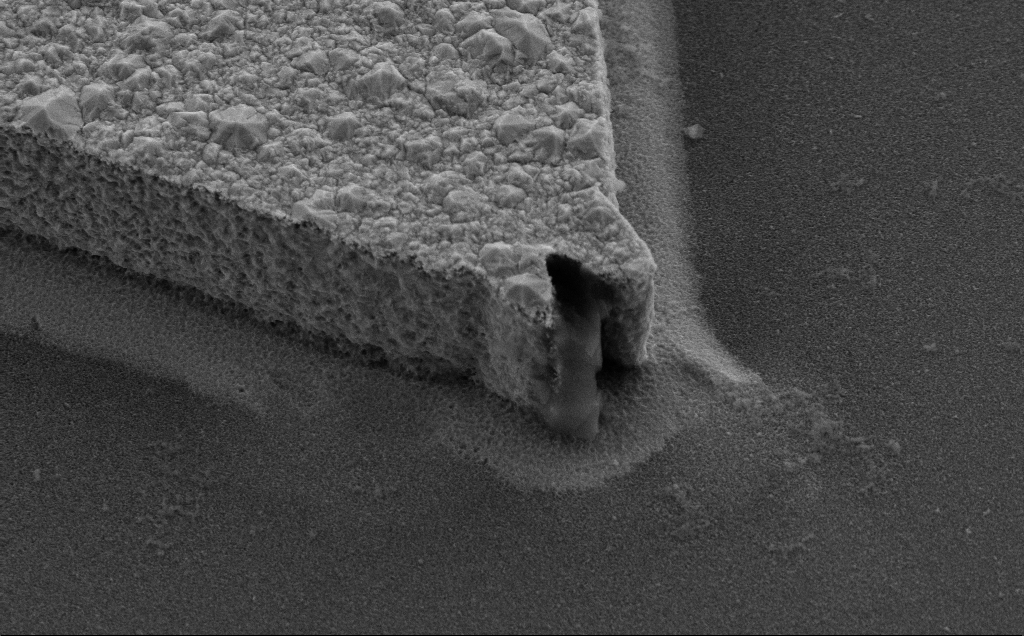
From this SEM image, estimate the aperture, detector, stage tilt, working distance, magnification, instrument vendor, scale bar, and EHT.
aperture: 30 µm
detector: SE2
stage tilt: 35°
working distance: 8 mm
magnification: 16.59 K X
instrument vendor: Zeiss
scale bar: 2000 nm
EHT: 10 kV